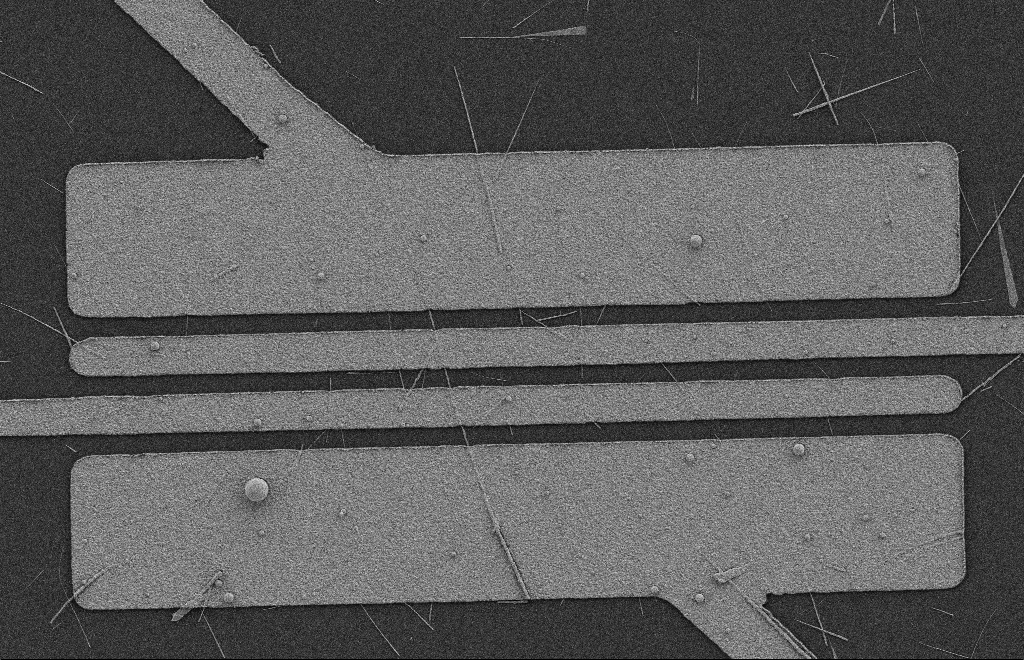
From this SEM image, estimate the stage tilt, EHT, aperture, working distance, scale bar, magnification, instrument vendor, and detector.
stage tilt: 0°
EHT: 2 kV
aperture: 20 µm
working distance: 9 mm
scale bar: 2000 nm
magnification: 5.37 K X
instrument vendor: Zeiss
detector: SE2